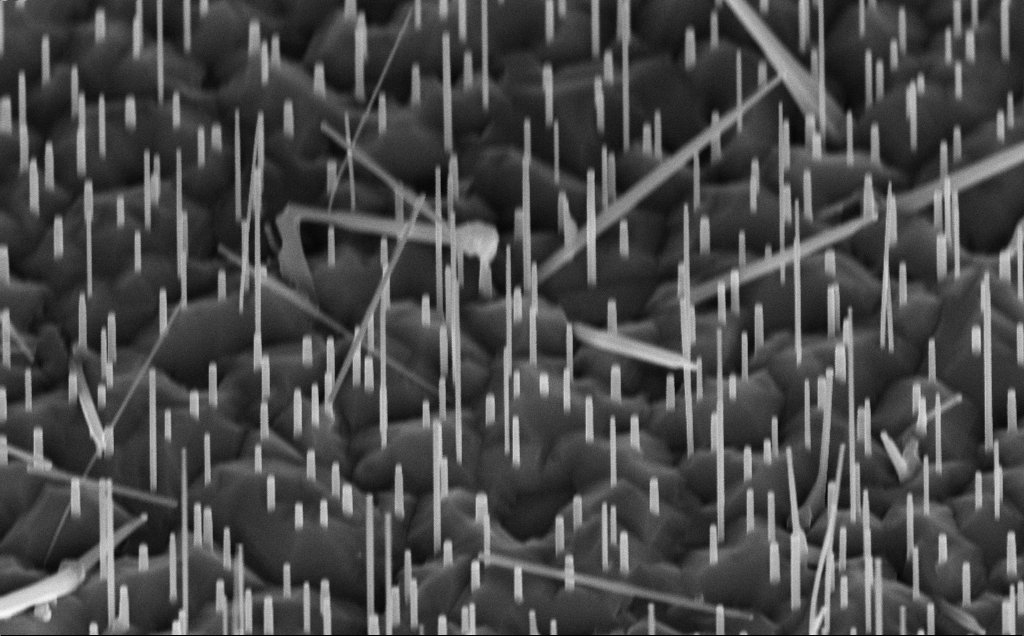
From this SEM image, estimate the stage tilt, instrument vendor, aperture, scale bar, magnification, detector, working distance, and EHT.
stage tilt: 45°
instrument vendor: Zeiss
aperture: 30 µm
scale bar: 1000 nm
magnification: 48.37 K X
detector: InLens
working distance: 7 mm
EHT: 10 kV